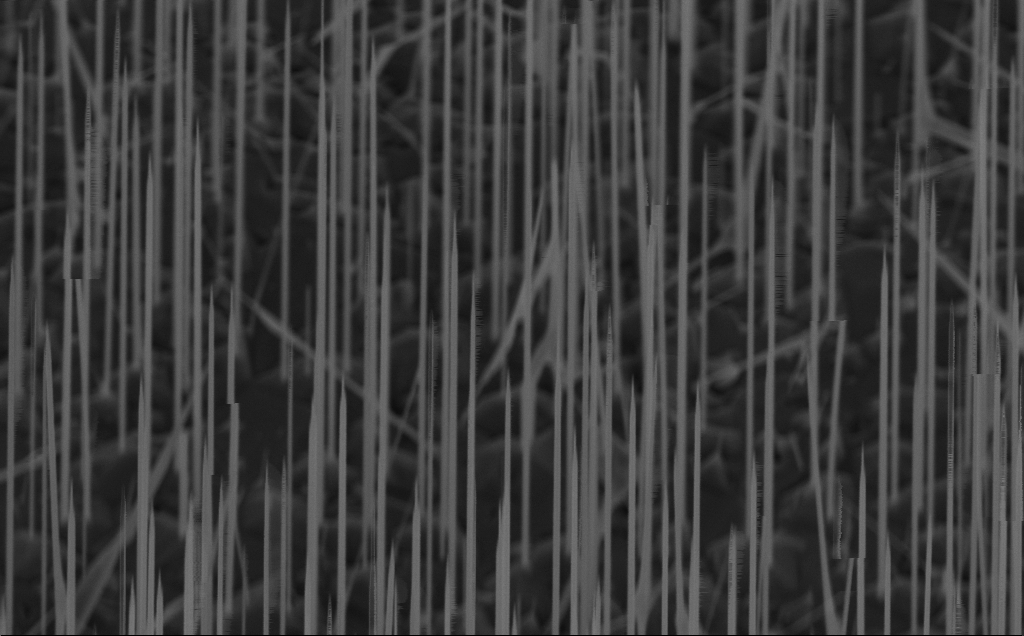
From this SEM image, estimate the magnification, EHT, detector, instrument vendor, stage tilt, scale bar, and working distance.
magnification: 40 K X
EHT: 5 kV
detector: InLens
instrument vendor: Zeiss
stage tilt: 45°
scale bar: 1000 nm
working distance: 8 mm